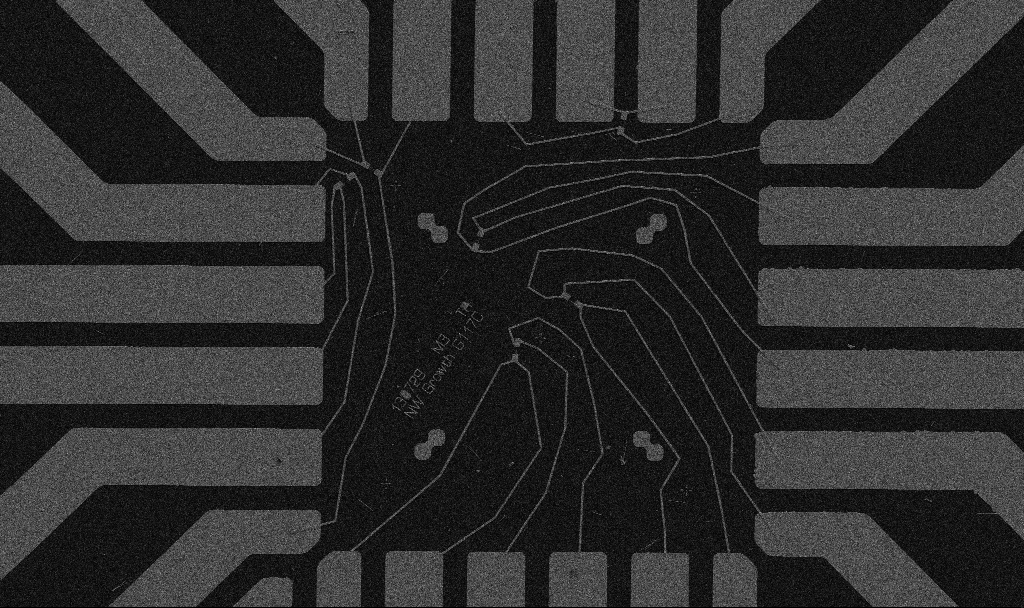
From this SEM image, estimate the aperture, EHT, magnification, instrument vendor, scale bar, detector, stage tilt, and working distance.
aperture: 30 µm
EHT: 5 kV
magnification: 1 K X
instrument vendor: Zeiss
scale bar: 20000 nm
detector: SE2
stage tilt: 0°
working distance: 10.7 mm